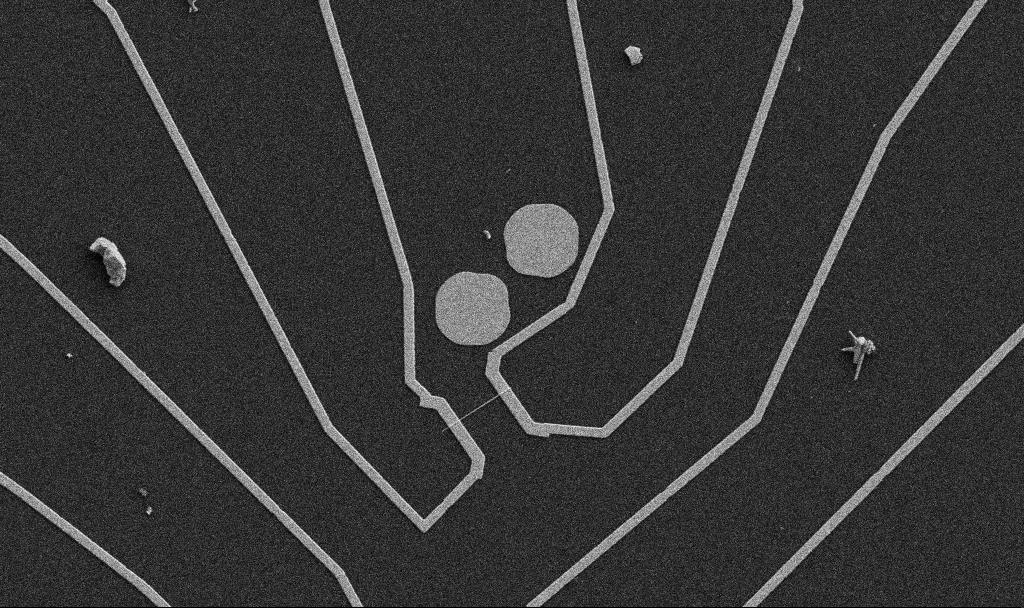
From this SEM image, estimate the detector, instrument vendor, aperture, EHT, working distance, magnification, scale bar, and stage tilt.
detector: SE2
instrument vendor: Zeiss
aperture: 30 µm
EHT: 5 kV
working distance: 10.7 mm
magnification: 5 K X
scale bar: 10000 nm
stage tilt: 0°